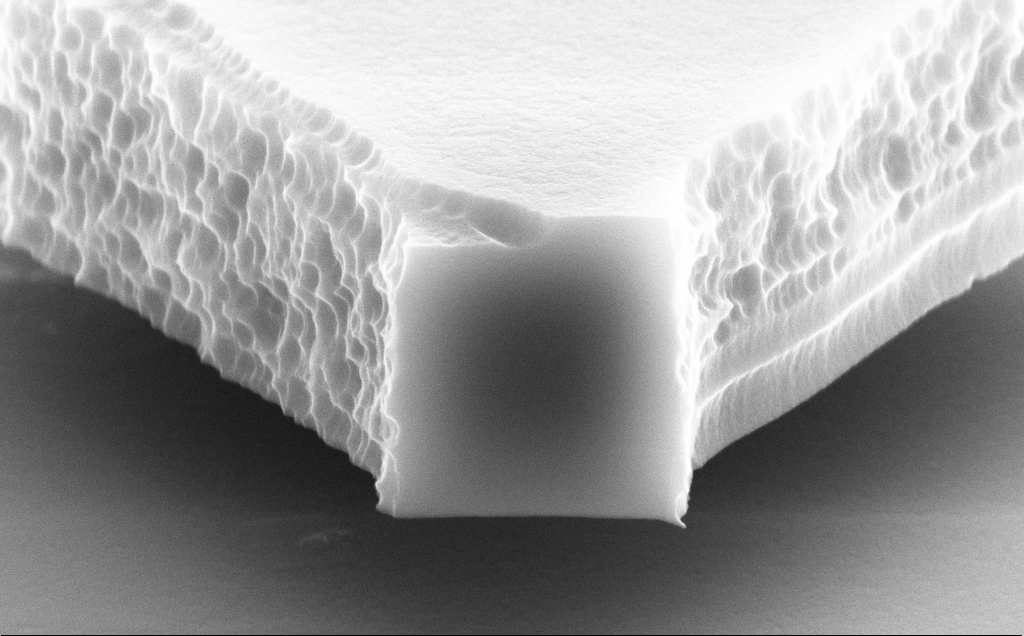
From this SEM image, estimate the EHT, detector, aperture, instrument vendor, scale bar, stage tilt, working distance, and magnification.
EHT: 10 kV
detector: SE2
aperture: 30 µm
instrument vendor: Zeiss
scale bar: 1000 nm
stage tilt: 70°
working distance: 9 mm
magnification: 47.87 K X